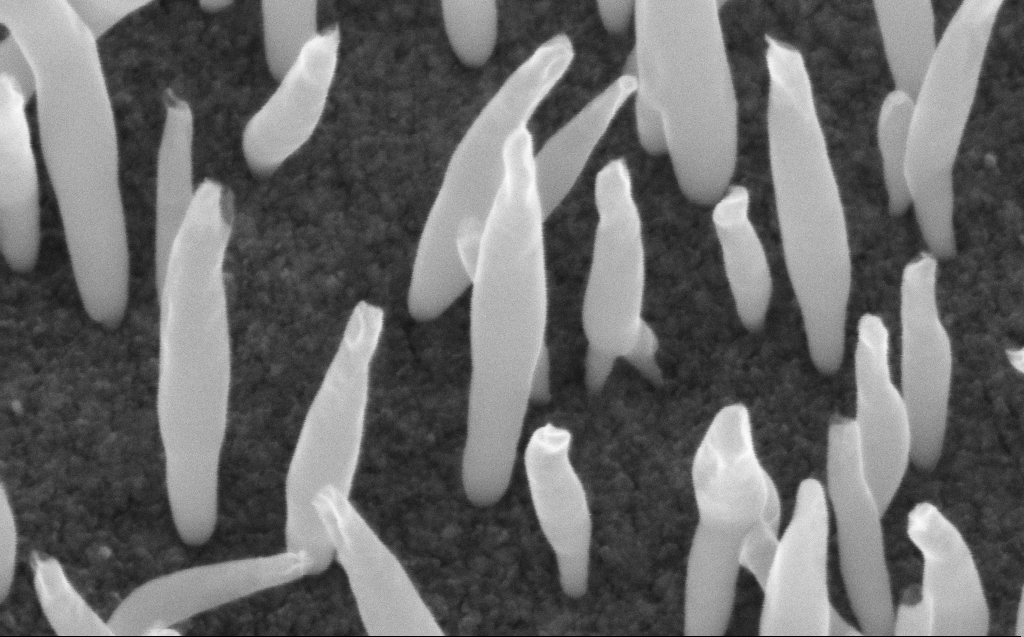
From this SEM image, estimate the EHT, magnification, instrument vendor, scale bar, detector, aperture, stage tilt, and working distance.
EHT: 10 kV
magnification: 200 K X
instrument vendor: Zeiss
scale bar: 100 nm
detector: InLens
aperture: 30 µm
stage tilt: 45°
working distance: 6 mm